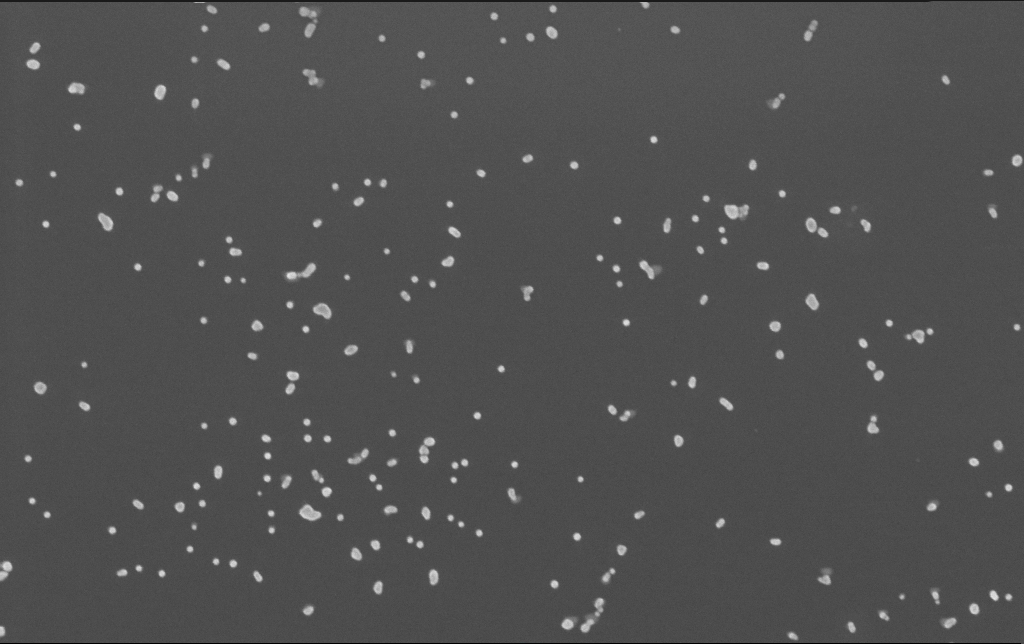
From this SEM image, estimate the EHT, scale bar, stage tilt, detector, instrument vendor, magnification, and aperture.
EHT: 10 kV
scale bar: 200 nm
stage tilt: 0°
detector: InLens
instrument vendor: Zeiss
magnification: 100 K X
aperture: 30 µm